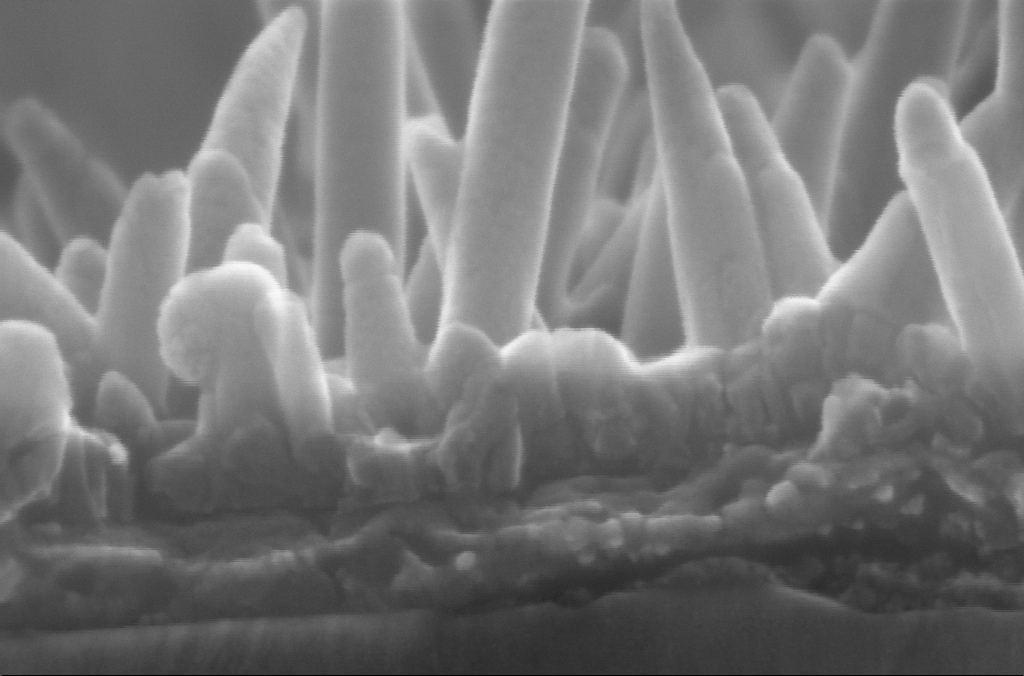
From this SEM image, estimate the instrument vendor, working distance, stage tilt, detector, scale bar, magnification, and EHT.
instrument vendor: Zeiss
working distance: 2.6 mm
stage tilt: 4°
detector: InLens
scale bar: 100 nm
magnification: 543.47 K X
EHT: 10 kV